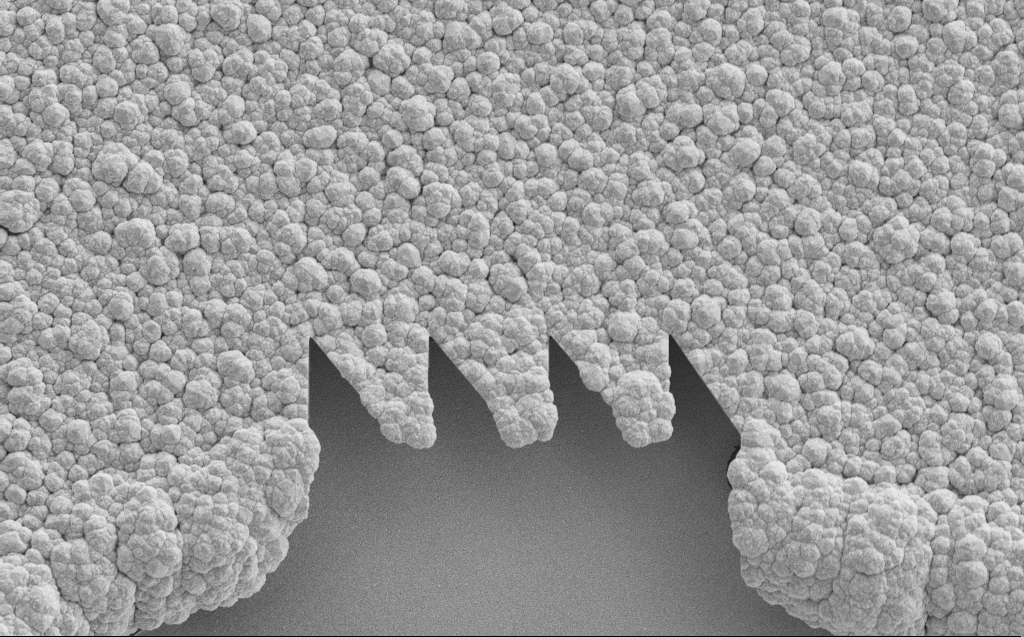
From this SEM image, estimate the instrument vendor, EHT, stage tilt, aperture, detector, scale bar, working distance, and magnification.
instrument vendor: Zeiss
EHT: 10 kV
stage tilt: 0°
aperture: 30 µm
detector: SE2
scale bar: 10000 nm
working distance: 7 mm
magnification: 3.82 K X